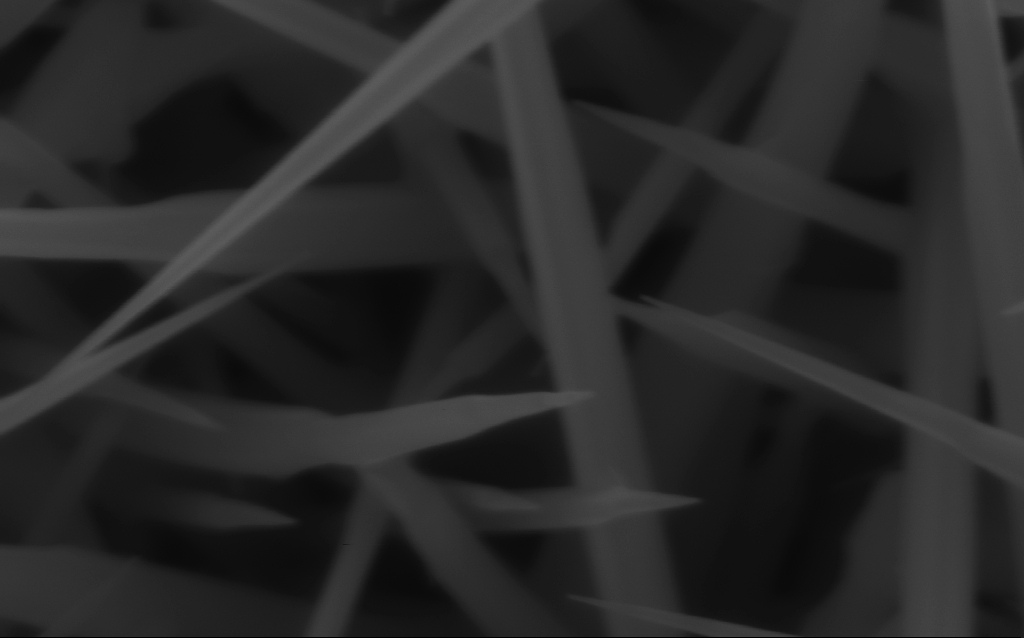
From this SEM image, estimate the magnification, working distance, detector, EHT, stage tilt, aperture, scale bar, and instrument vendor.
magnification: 150 K X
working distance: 6 mm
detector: InLens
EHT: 10 kV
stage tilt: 0°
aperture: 30 µm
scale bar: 200 nm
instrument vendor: Zeiss